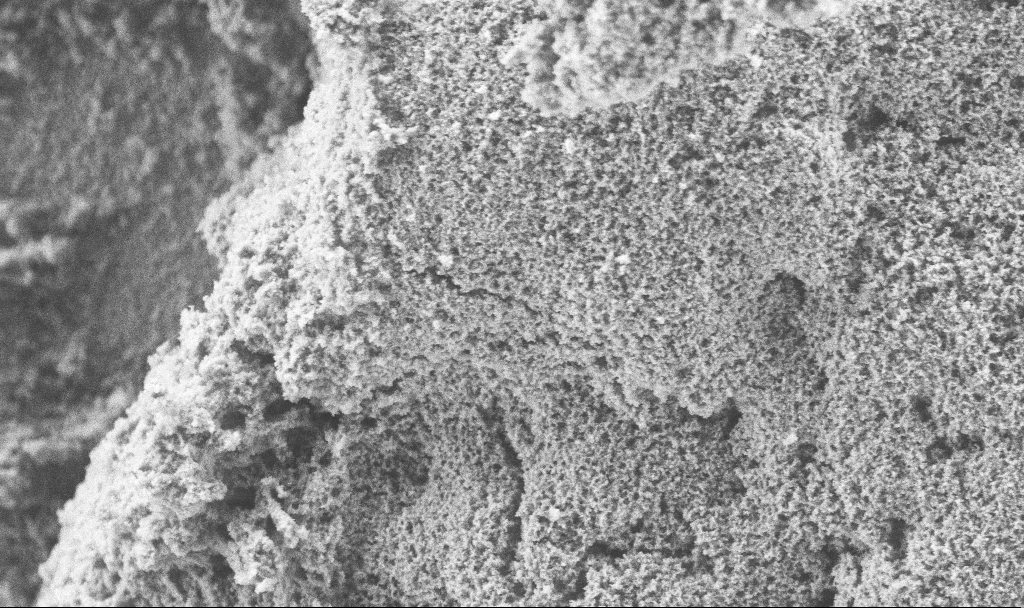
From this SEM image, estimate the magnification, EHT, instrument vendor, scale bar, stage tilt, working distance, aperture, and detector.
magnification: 21.8 K X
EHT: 3 kV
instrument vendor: Zeiss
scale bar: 2000 nm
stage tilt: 0°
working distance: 2.7 mm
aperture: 30 µm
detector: SE2